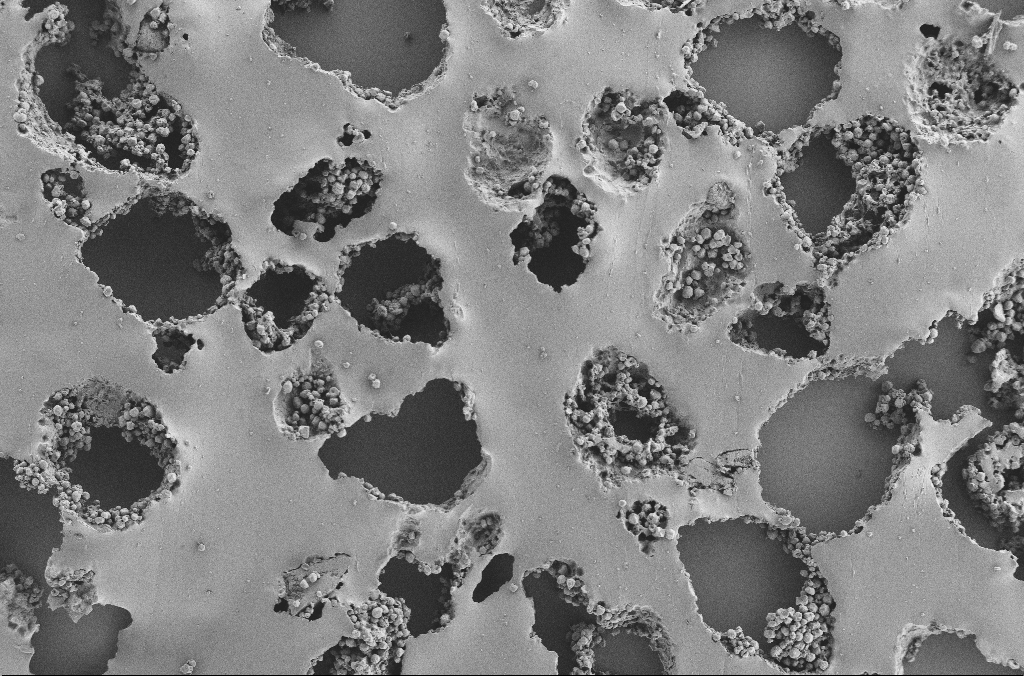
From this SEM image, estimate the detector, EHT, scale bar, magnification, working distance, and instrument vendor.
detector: SE2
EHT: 2 kV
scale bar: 100000 nm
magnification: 0.25 K X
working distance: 3.7 mm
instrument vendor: Zeiss